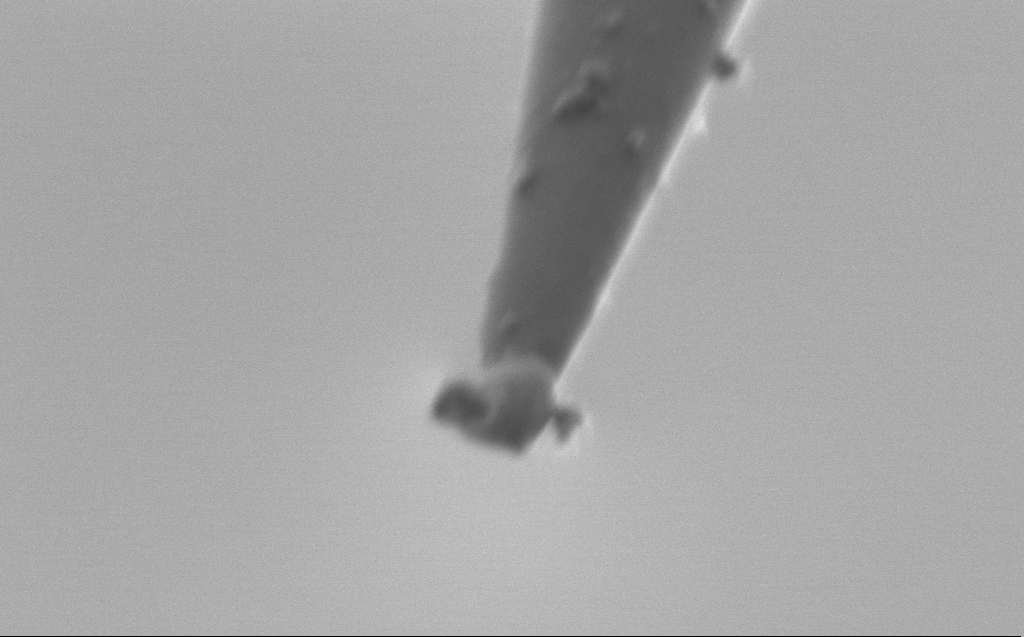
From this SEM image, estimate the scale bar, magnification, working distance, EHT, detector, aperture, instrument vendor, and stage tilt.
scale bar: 200 nm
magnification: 100 K X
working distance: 5 mm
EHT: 1 kV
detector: SE2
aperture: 30 µm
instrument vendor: Zeiss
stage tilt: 45°